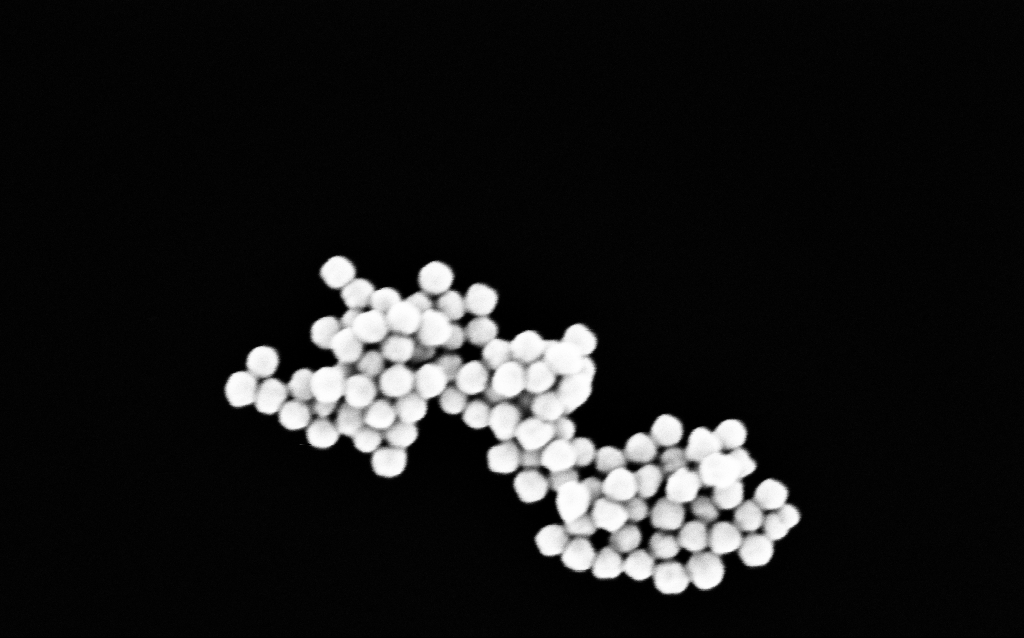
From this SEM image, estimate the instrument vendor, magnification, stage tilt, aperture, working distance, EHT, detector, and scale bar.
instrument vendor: Zeiss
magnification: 200 K X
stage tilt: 0°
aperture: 30 µm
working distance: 3.1 mm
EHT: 5 kV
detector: InLens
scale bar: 100 nm